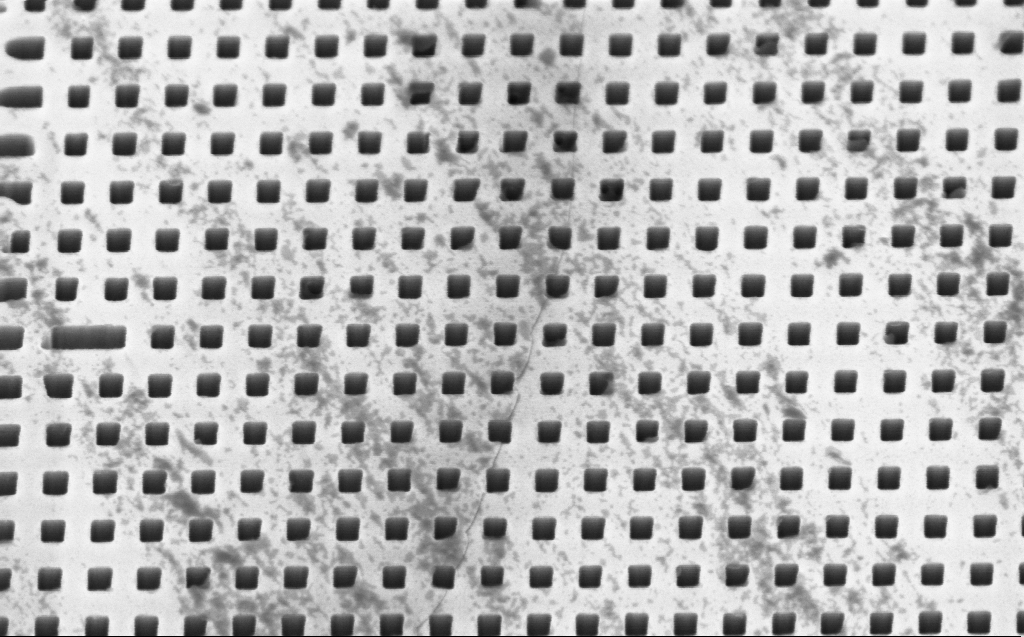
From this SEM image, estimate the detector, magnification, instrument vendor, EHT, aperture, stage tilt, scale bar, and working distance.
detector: InLens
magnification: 36.17 K X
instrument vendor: Zeiss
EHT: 3 kV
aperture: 30 µm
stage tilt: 45°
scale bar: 1000 nm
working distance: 6 mm